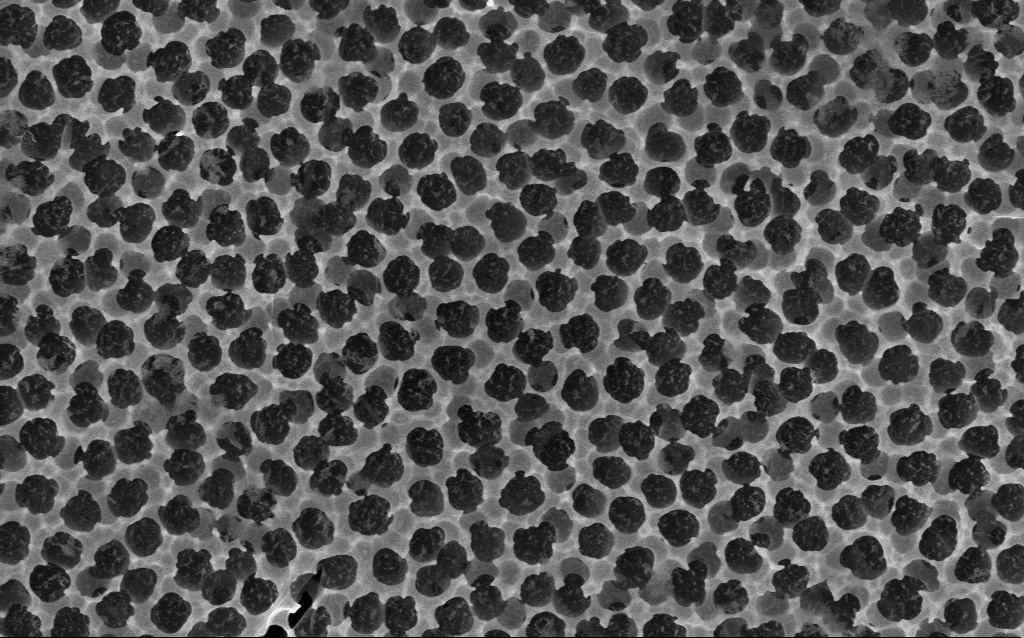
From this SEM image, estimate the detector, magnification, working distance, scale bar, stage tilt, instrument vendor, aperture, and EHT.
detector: InLens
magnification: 56.34 K X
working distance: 3.9 mm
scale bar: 1000 nm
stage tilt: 0.1°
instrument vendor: Zeiss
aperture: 30 µm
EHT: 5 kV